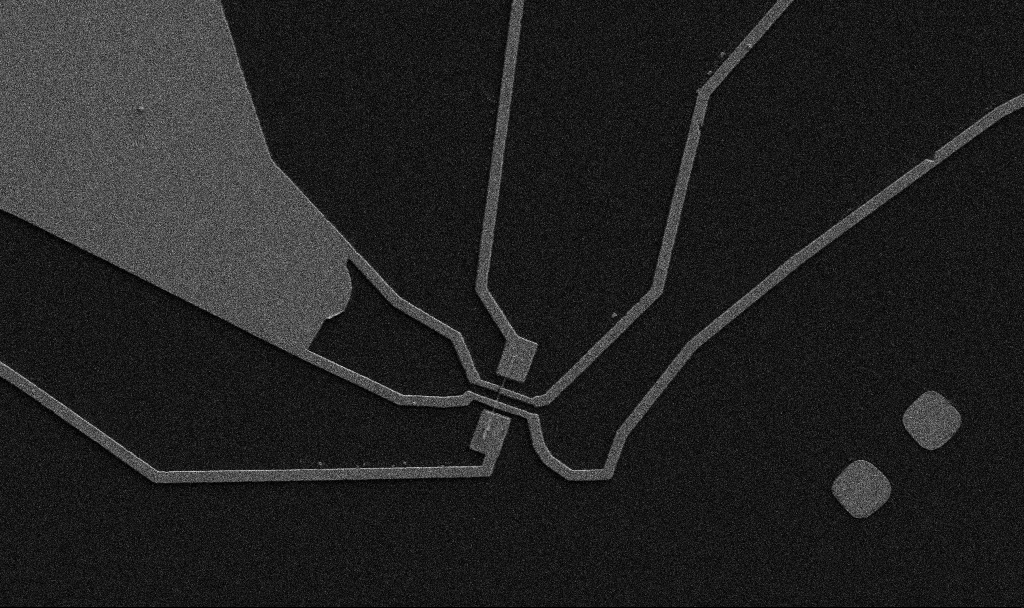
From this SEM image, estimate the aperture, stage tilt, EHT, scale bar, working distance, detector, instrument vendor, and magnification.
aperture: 30 µm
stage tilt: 0°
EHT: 5 kV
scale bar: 10000 nm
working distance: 10.7 mm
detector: SE2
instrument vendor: Zeiss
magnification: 5 K X